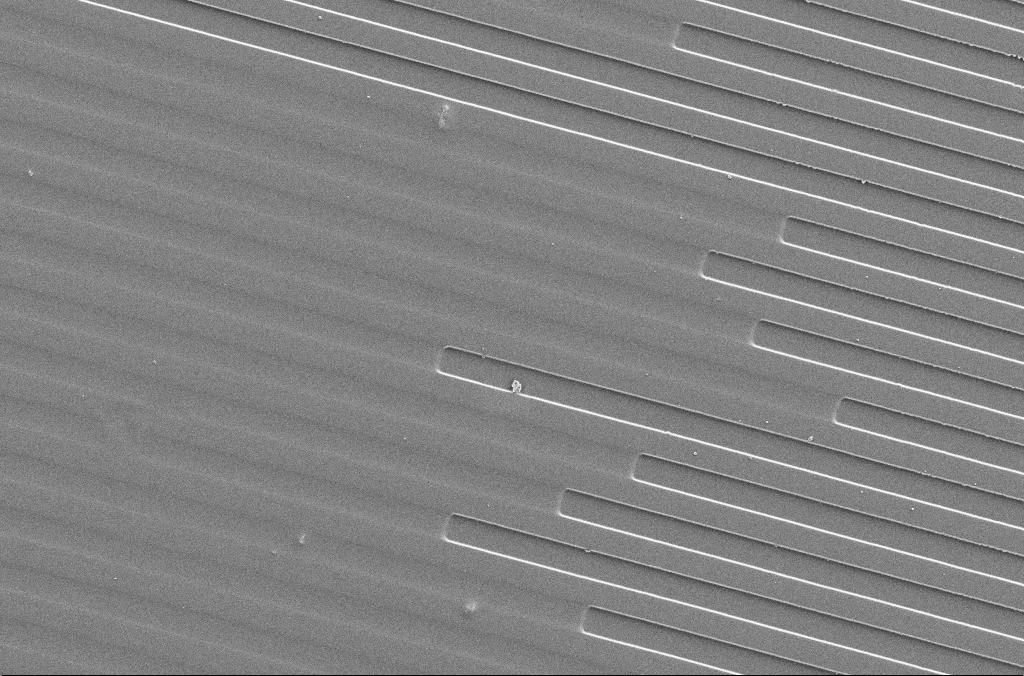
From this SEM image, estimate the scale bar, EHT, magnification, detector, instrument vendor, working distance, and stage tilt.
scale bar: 20000 nm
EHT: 5 kV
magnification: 1 K X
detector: SE2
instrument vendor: Zeiss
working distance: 4.1 mm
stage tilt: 0°